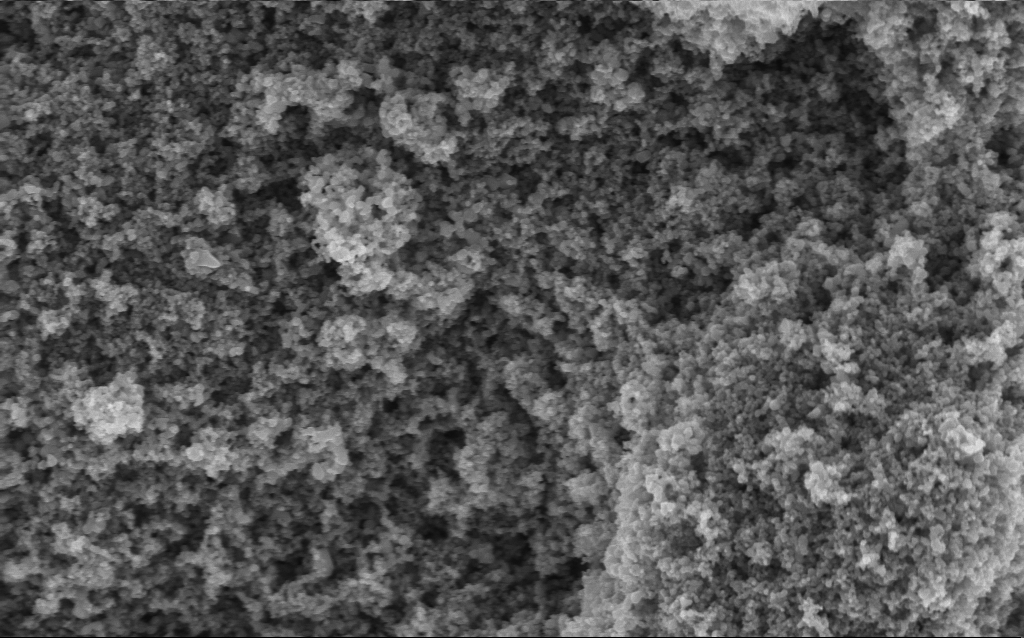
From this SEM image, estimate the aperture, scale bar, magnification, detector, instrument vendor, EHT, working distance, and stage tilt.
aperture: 30 µm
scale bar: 1000 nm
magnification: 68.59 K X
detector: InLens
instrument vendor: Zeiss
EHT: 5 kV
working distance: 4.2 mm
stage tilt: -0°